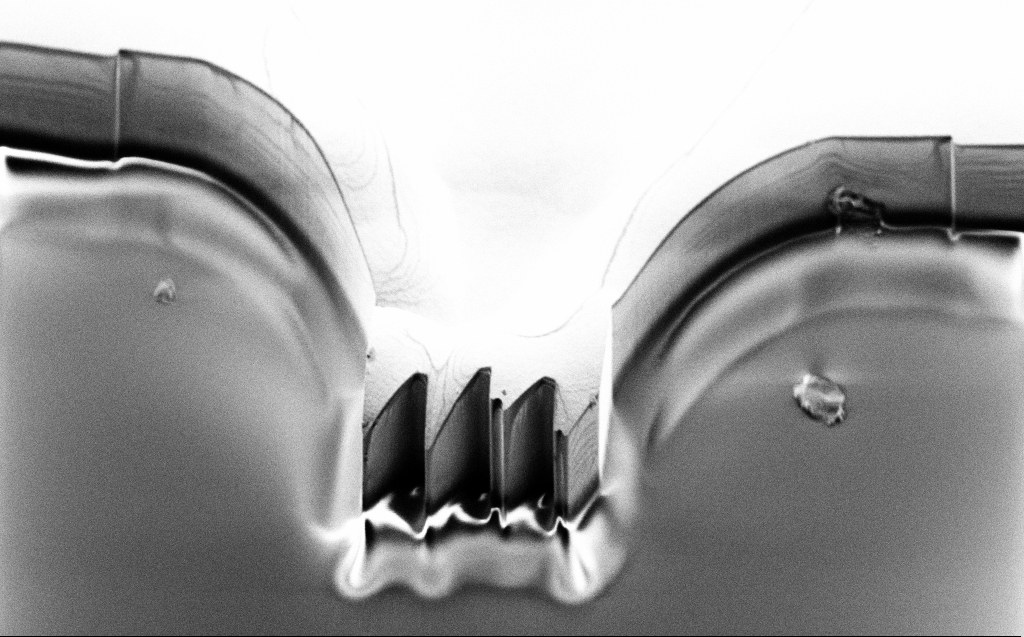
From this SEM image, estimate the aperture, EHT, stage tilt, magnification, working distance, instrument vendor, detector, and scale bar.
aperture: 30 µm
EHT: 3 kV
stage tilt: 45.1°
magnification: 2.07 K X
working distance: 10 mm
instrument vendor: Zeiss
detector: SE2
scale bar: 20000 nm